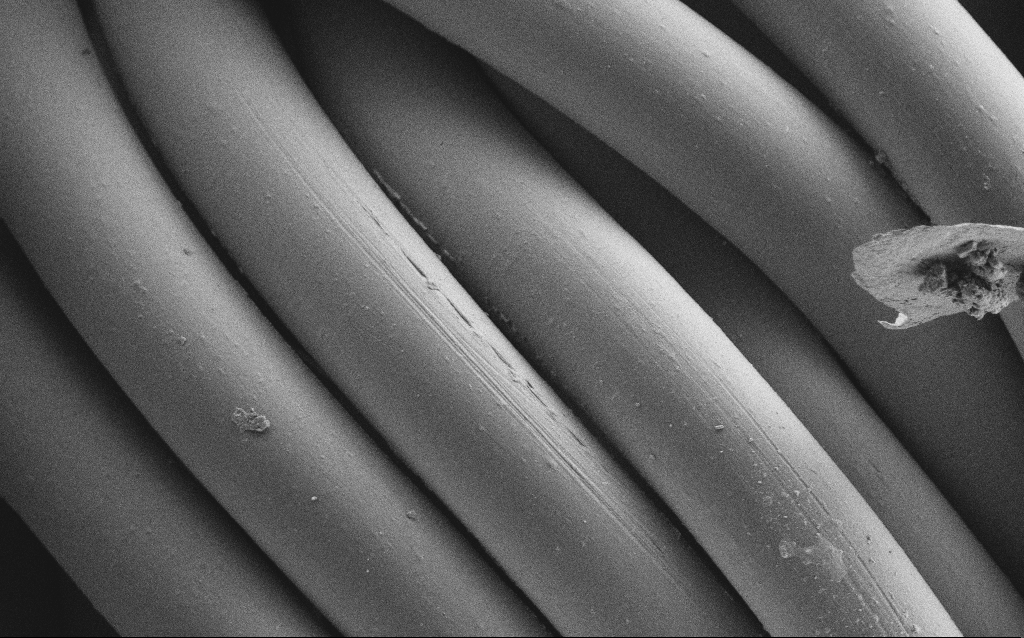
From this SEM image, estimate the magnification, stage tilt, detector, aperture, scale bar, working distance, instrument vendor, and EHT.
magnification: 2.49 K X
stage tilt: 0°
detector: SE2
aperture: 30 µm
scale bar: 20000 nm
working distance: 4 mm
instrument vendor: Zeiss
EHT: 1 kV